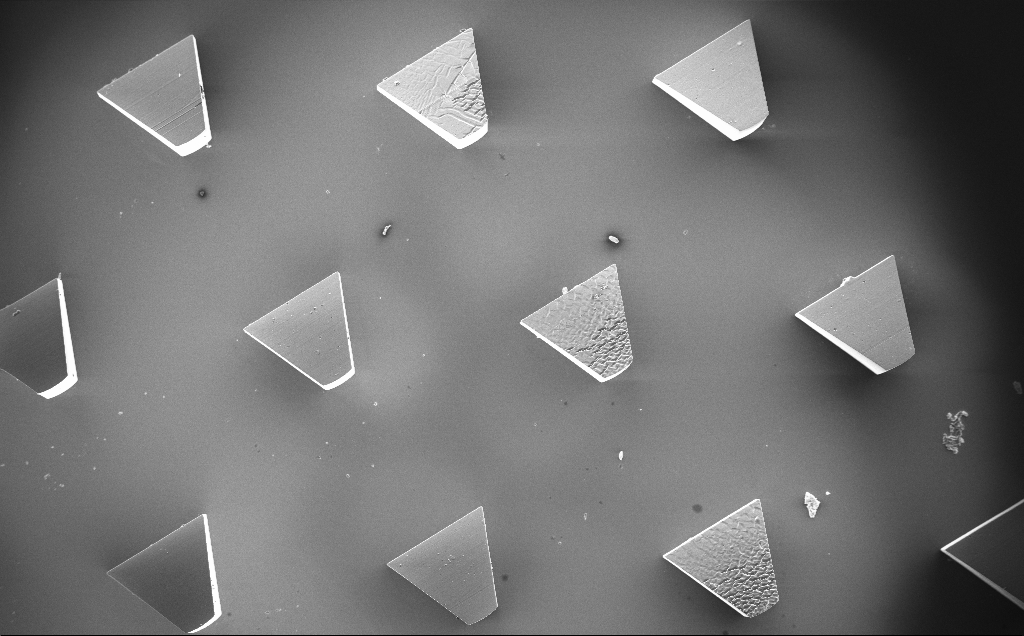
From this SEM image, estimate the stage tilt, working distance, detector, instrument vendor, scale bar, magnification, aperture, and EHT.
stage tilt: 0°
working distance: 10 mm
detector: InLens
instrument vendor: Zeiss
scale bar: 200000 nm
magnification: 0.104 K X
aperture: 30 µm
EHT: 10 kV